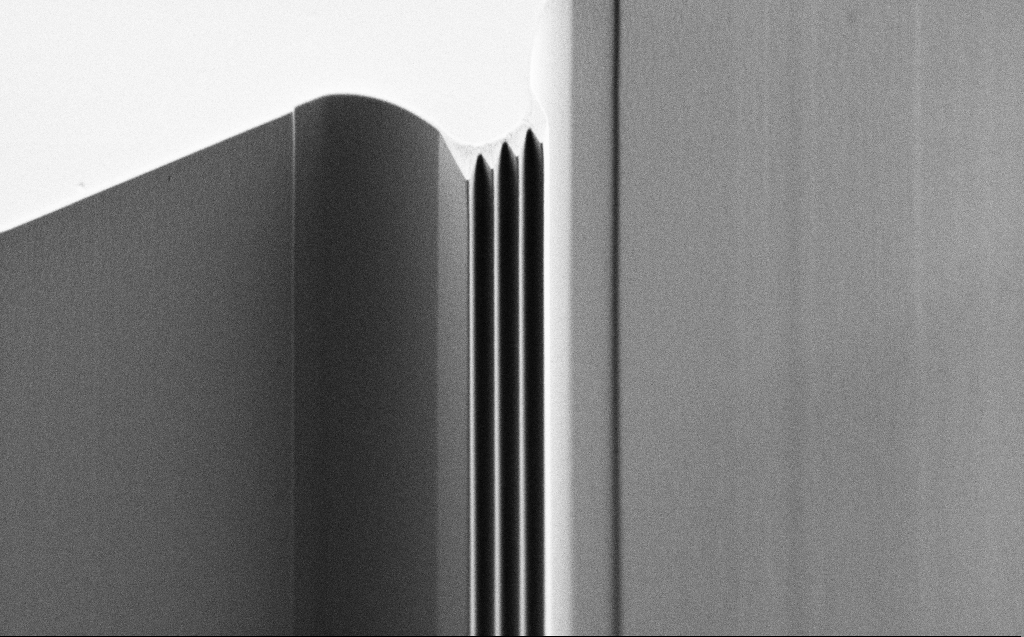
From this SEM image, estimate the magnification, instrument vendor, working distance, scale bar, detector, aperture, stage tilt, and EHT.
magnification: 0.893 K X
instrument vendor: Zeiss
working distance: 5 mm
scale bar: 20000 nm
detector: SE2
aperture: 30 µm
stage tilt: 45°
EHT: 0.9 kV